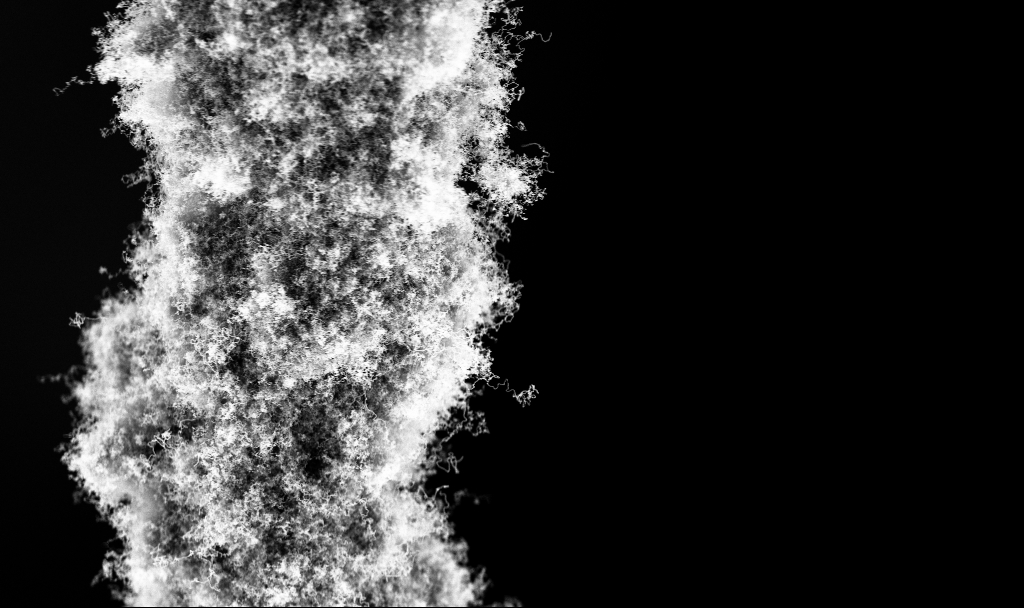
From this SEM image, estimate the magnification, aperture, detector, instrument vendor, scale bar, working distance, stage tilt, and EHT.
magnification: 10 K X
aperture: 30 µm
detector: InLens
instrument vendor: Zeiss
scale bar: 2000 nm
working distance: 2.7 mm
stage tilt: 0°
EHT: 3 kV